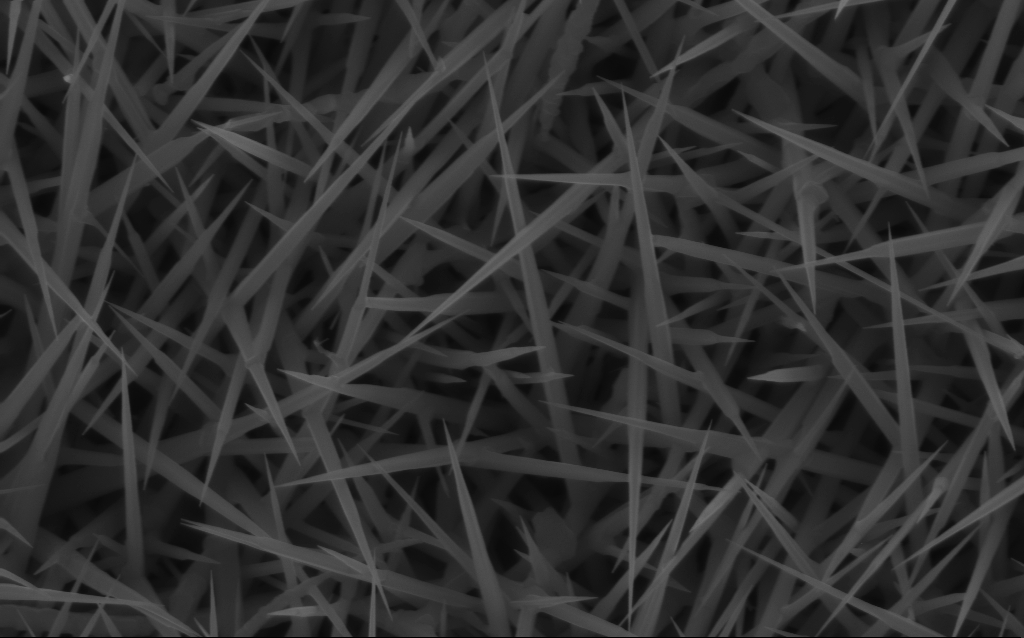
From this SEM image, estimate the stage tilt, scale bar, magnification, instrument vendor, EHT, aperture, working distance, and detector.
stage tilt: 0°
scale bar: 1000 nm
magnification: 40 K X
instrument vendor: Zeiss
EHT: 10 kV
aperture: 30 µm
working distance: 6 mm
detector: InLens